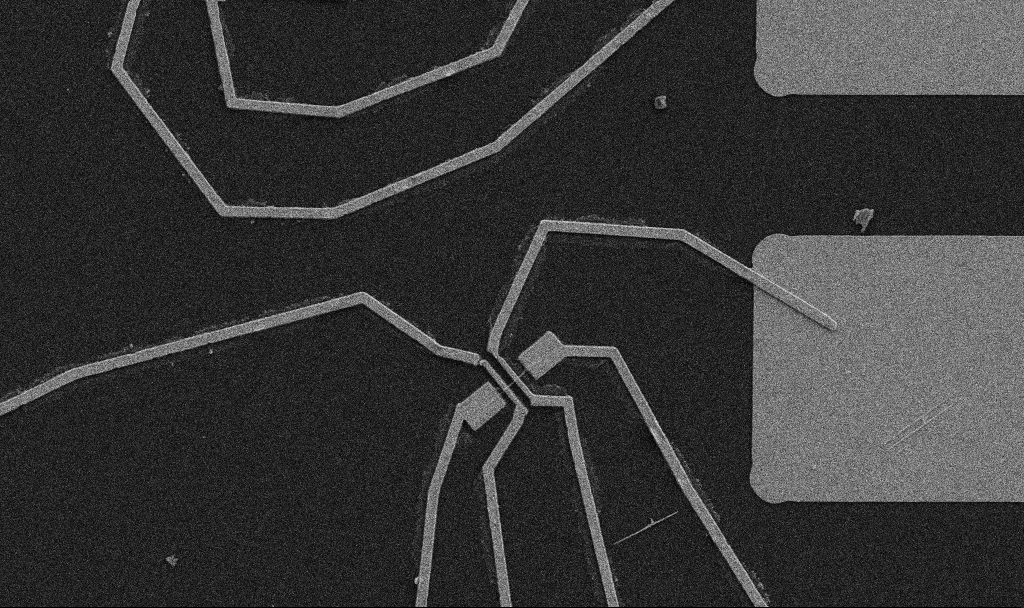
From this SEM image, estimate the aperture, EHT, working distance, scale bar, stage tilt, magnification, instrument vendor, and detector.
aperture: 30 µm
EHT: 5 kV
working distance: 10.7 mm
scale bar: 10000 nm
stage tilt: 0°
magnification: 5 K X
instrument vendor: Zeiss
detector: SE2